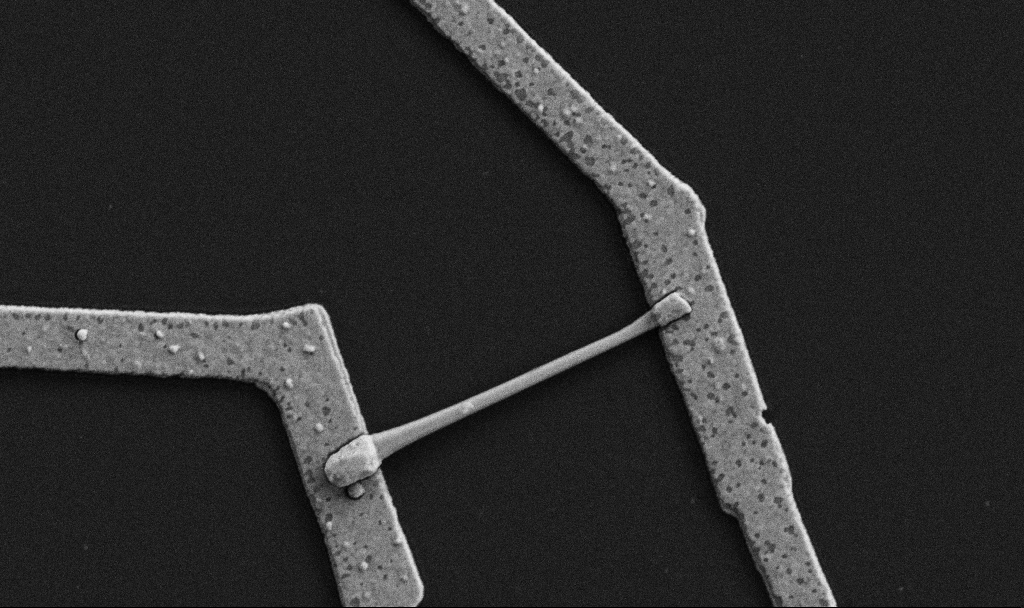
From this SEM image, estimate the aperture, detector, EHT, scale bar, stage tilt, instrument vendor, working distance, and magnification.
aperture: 30 µm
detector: SE2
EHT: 5 kV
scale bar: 1000 nm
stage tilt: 0°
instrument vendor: Zeiss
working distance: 8.7 mm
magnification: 30 K X